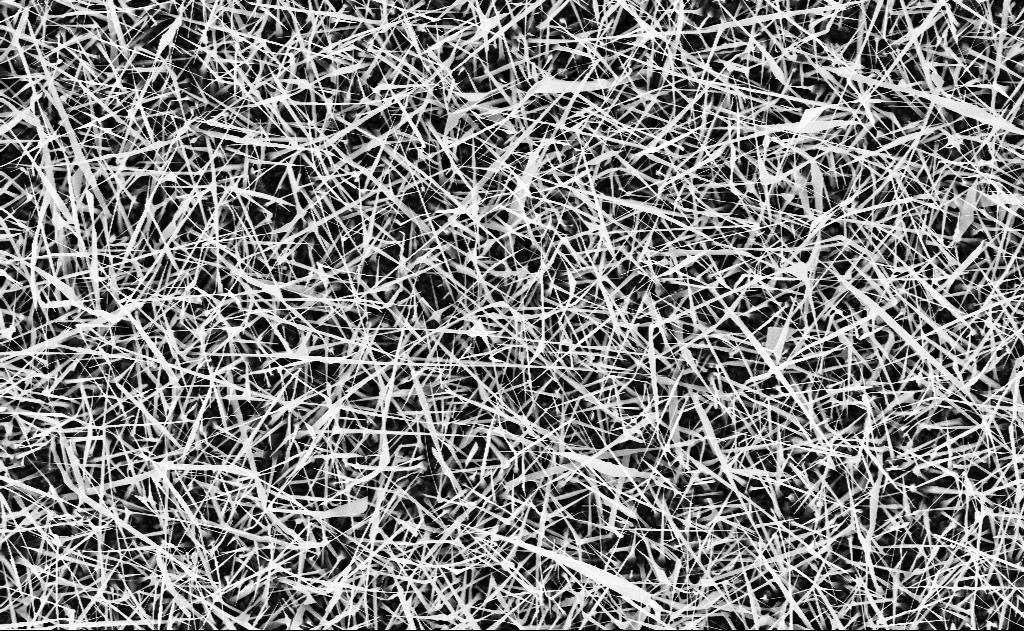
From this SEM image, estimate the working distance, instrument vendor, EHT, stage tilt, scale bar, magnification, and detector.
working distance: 15 mm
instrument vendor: Zeiss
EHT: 10 kV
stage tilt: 0°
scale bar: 2000 nm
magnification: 10 K X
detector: InLens